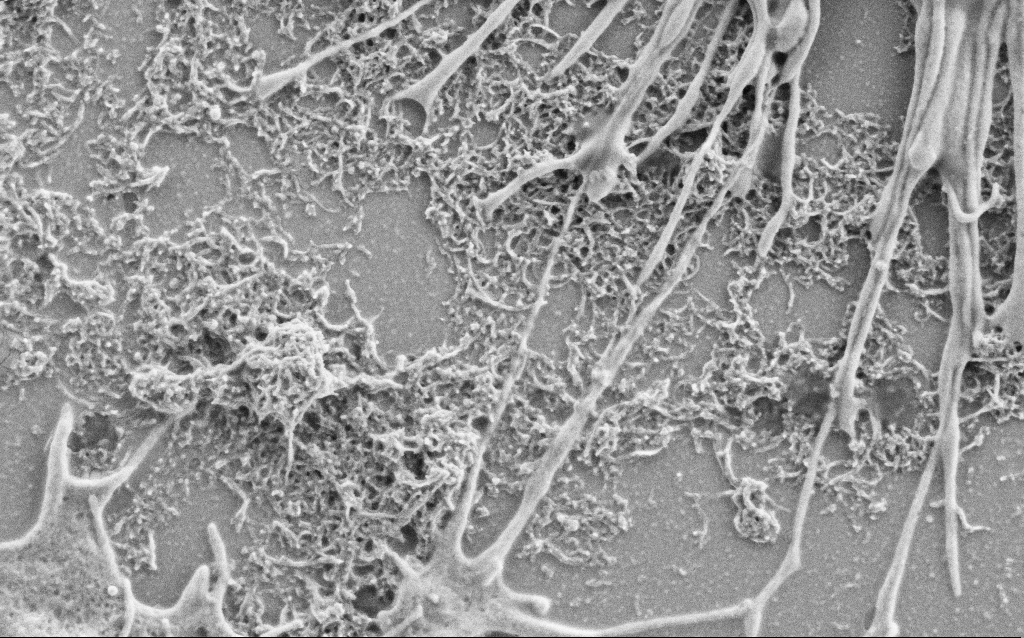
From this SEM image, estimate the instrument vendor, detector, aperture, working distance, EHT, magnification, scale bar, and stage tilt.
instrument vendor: Zeiss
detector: SE2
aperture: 30 µm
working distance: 6.9 mm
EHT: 2 kV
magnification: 25 K X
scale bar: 1000 nm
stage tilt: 0°